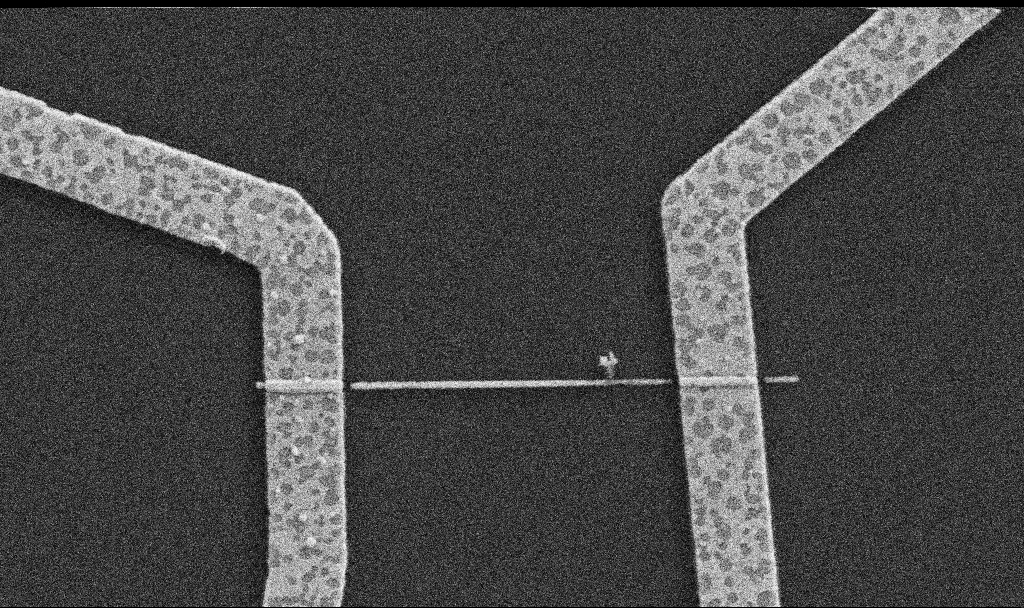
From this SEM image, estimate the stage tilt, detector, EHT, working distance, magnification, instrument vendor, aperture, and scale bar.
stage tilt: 0°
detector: SE2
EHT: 5 kV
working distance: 8.7 mm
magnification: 30 K X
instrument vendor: Zeiss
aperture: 30 µm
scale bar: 1000 nm